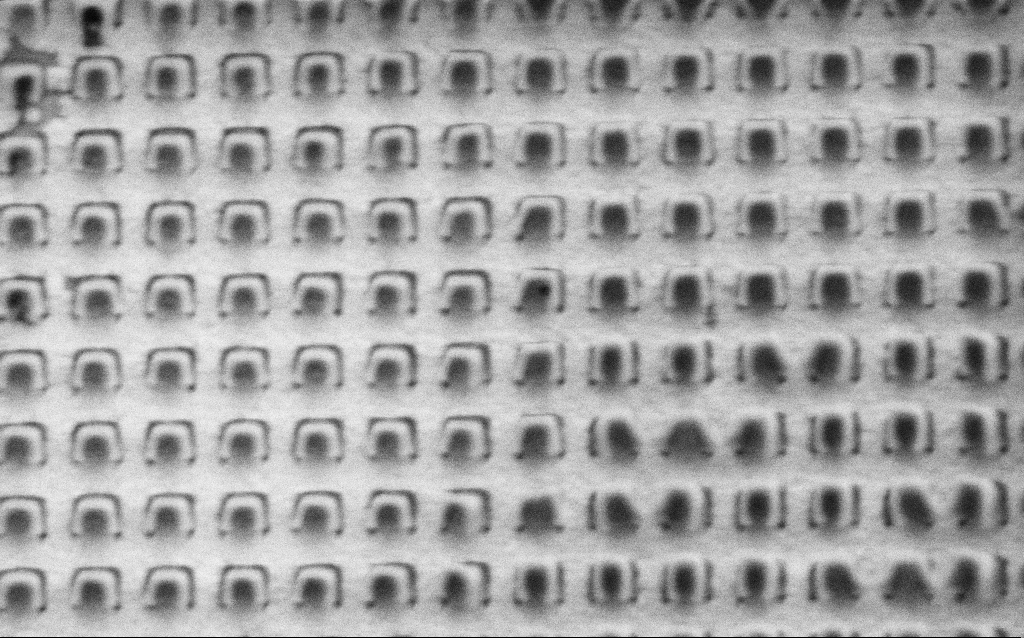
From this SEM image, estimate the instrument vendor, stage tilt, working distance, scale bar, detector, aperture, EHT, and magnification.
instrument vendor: Zeiss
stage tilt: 0°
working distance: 9.3 mm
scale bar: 1000 nm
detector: SE2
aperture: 30 µm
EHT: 1.5 kV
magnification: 58.05 K X